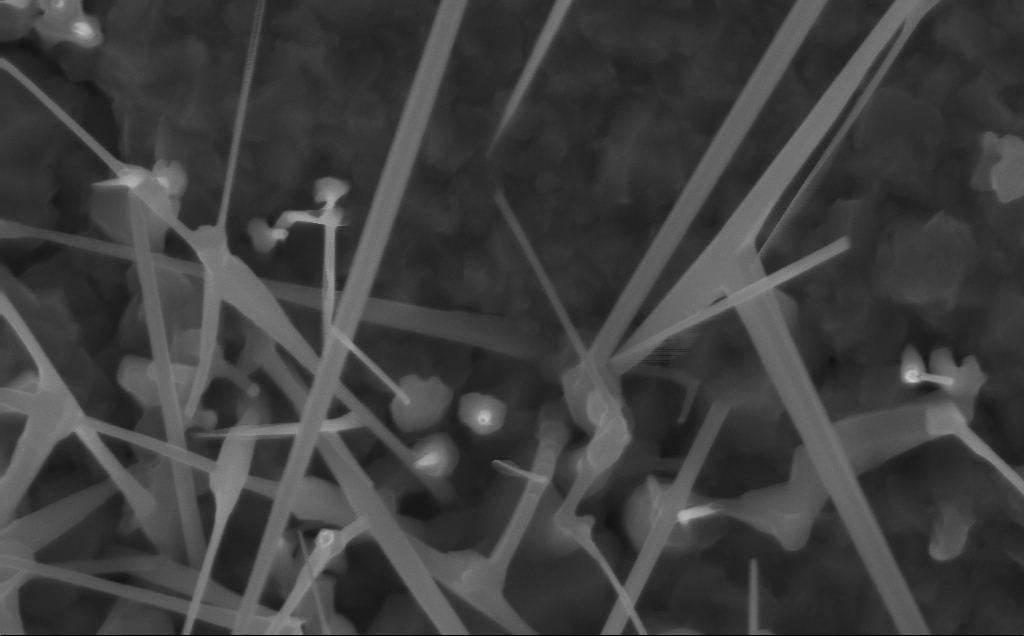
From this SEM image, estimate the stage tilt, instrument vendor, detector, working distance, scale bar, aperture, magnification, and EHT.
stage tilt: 0°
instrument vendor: Zeiss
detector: InLens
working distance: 3 mm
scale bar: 200 nm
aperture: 30 µm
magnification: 80 K X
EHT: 10 kV